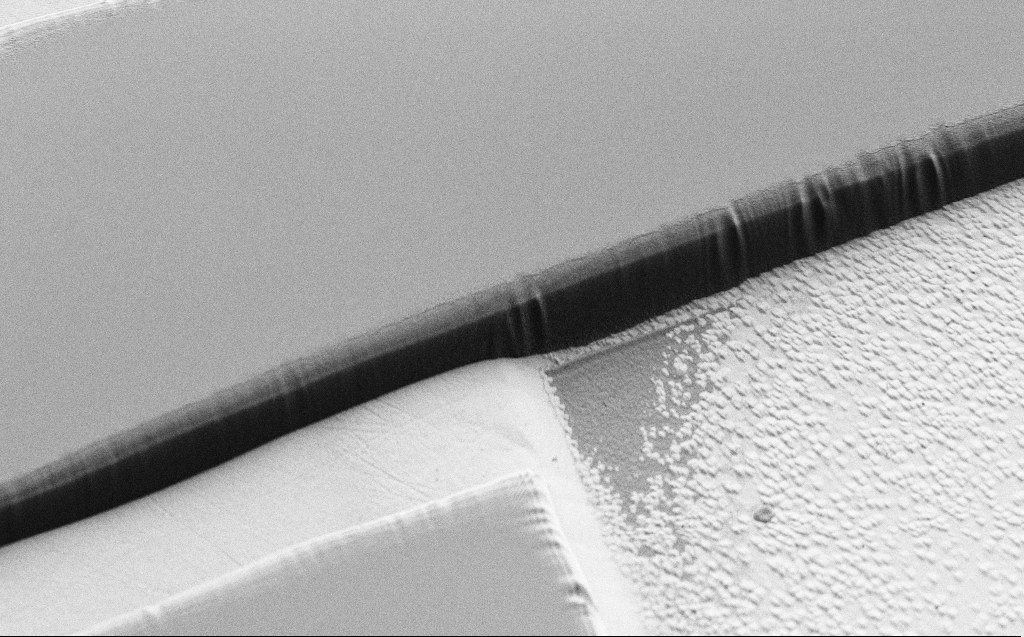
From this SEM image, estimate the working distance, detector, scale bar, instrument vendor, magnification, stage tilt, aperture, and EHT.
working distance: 4 mm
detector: SE2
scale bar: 20000 nm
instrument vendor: Zeiss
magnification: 2.03 K X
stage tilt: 45°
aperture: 30 µm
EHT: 1 kV